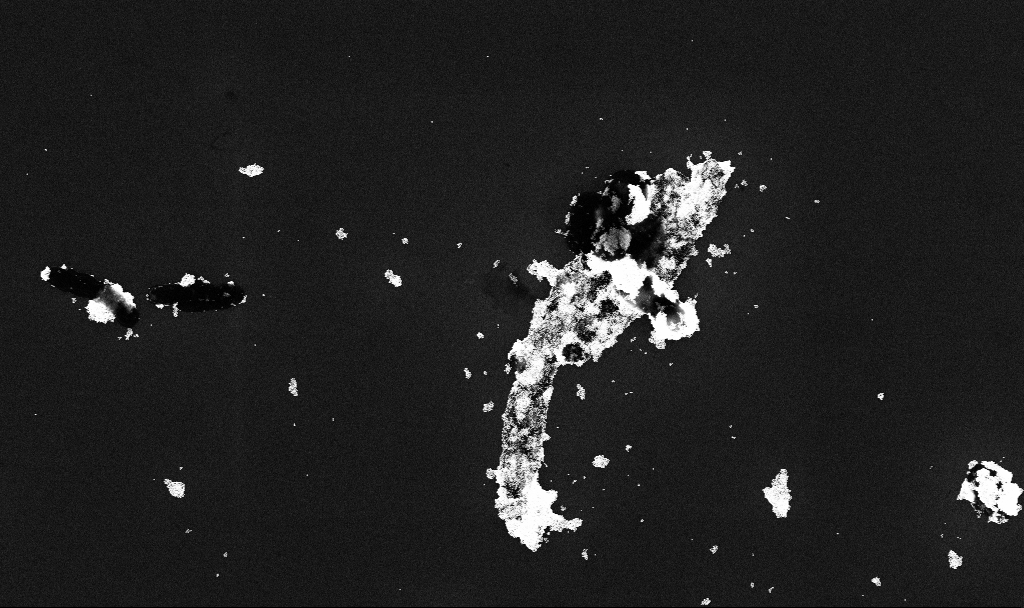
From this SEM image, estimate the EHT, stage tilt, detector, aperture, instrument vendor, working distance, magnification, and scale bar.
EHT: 10 kV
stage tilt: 0°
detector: InLens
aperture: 30 µm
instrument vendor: Zeiss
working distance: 3.3 mm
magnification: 21.11 K X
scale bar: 1000 nm